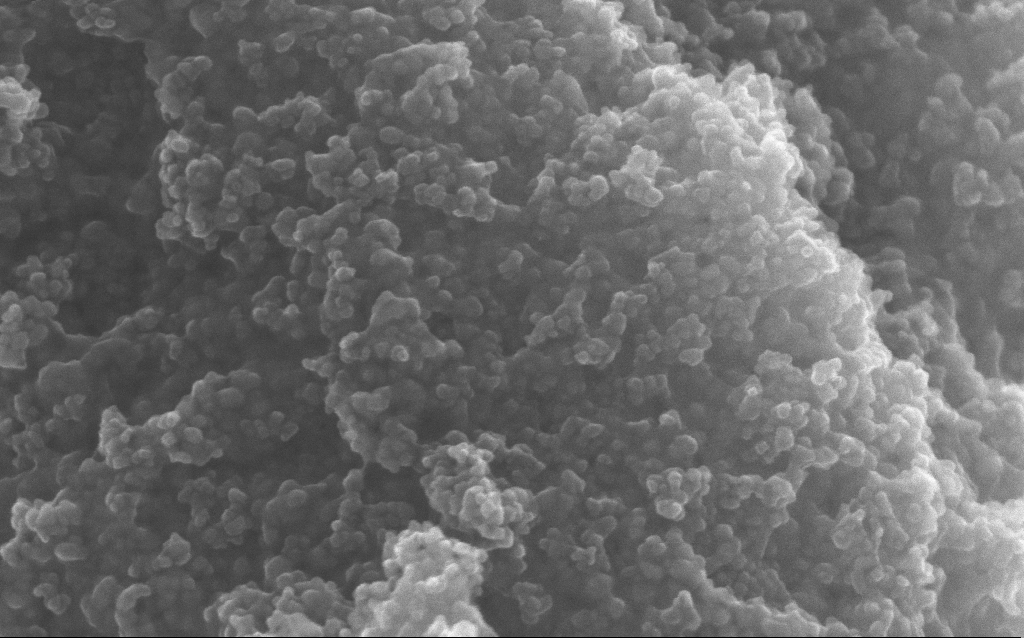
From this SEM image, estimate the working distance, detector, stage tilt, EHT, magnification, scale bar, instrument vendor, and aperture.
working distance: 2.7 mm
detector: InLens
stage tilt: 0°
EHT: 10 kV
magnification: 204.13 K X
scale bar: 100 nm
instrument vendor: Zeiss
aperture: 30 µm